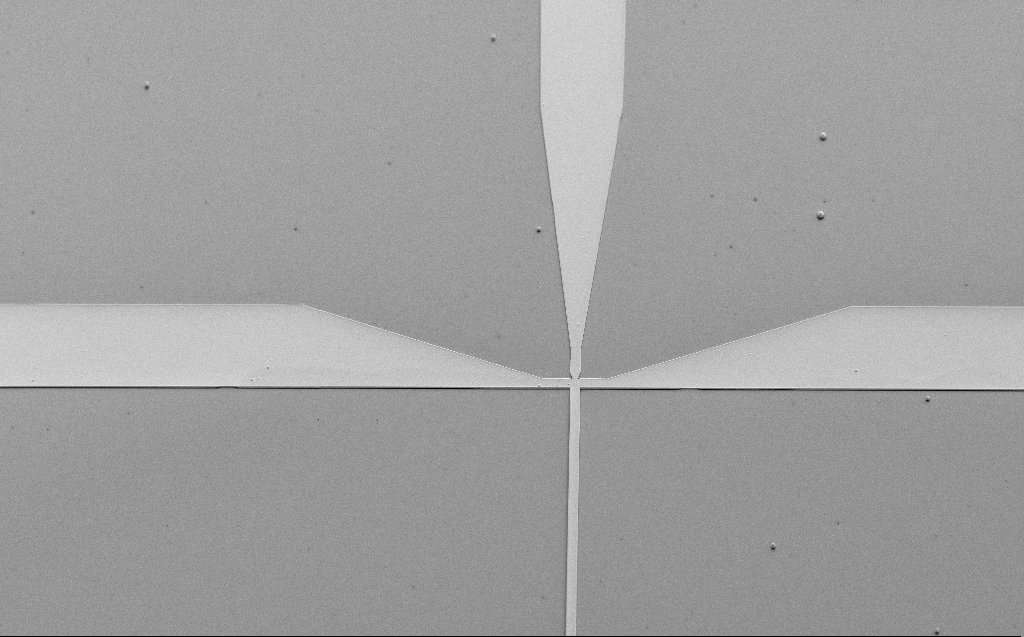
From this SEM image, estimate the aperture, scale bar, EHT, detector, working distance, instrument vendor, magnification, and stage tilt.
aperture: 30 µm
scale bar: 100000 nm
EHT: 5 kV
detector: SE2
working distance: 9 mm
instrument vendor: Zeiss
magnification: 0.3 K X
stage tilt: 45°